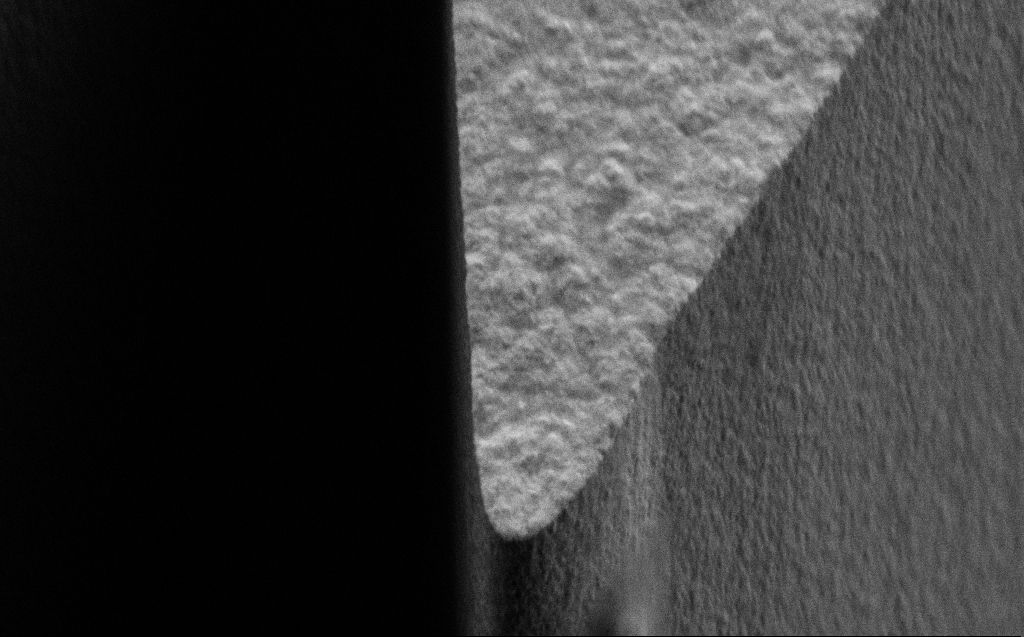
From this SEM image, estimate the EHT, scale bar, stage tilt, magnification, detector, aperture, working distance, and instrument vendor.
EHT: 3 kV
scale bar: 2000 nm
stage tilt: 45°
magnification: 36.06 K X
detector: SE2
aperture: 30 µm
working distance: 5 mm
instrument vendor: Zeiss